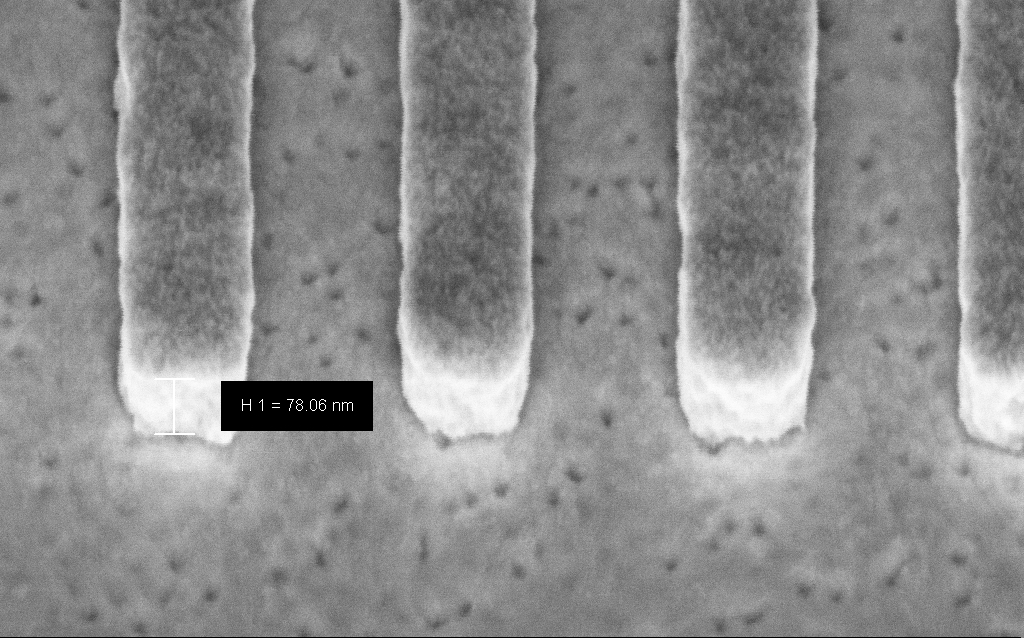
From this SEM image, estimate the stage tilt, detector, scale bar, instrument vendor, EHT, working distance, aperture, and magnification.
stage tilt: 45°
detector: InLens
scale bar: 200 nm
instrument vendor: Zeiss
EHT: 3 kV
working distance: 6.6 mm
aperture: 30 µm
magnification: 258.72 K X